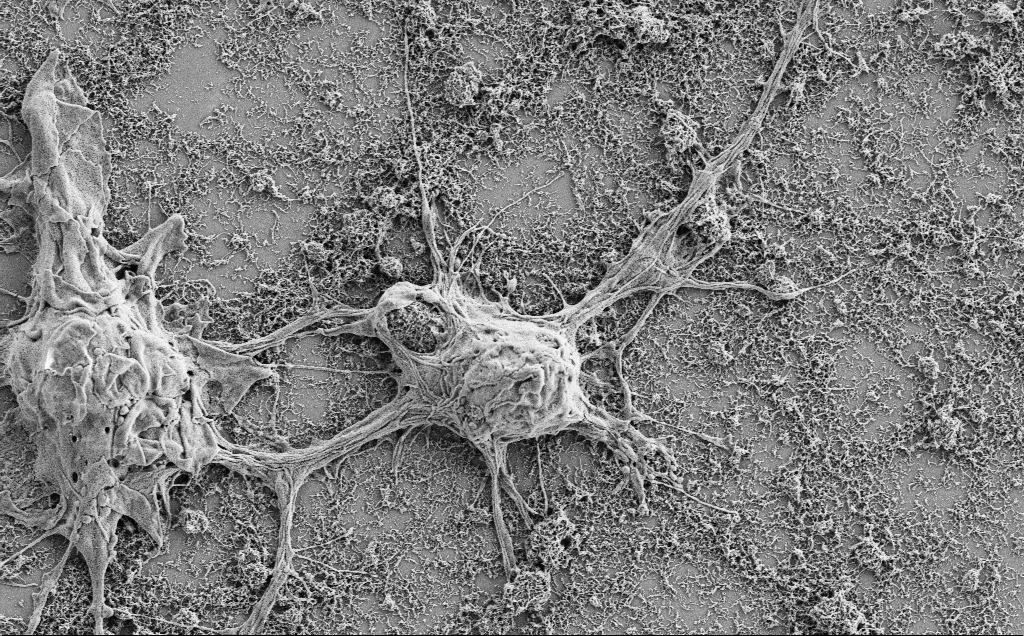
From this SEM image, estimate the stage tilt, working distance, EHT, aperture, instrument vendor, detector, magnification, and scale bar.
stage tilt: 0°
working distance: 7 mm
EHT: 2 kV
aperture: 30 µm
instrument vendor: Zeiss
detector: SE2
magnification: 7.5 K X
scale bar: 2000 nm